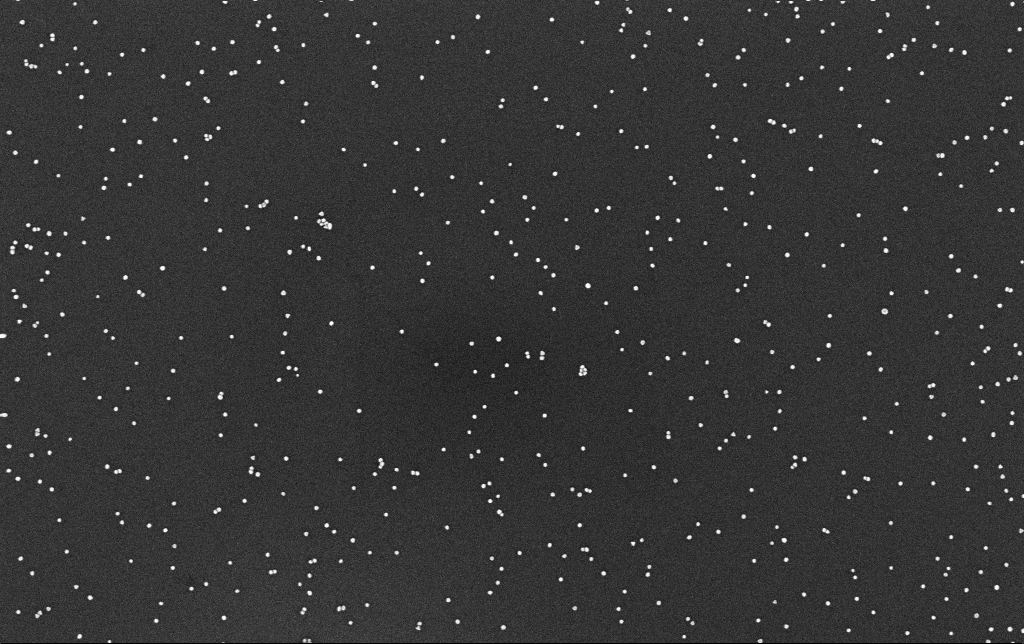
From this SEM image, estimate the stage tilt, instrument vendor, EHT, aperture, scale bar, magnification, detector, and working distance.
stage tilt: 0°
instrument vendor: Zeiss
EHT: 10 kV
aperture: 30 µm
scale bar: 200 nm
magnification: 100 K X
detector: InLens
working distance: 3.4 mm